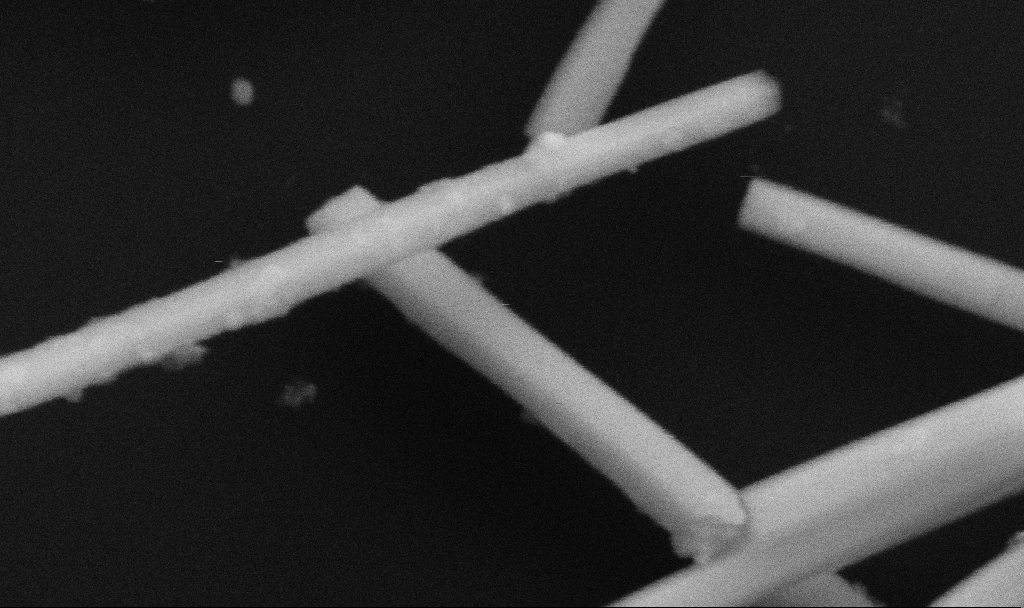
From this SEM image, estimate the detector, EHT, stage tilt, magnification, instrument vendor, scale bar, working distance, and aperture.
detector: SE2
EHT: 5 kV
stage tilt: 0°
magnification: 252.81 K X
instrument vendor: Zeiss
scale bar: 200 nm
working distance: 6.7 mm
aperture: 30 µm